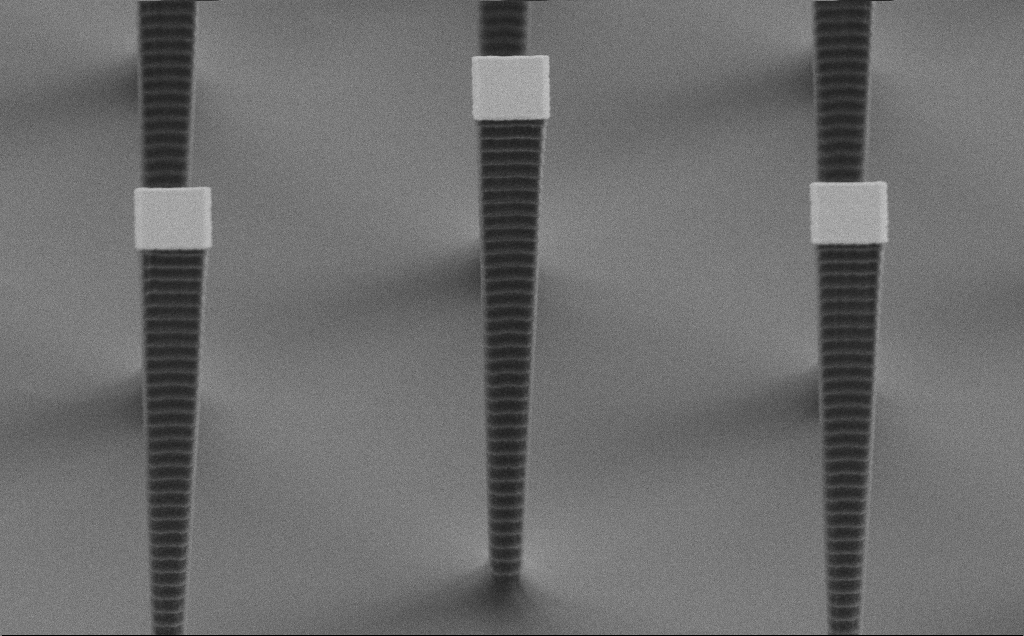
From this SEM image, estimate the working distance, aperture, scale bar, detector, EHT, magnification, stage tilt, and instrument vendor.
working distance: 7 mm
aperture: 30 µm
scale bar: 2000 nm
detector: SE2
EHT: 3 kV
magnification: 13.67 K X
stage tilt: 60°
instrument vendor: Zeiss